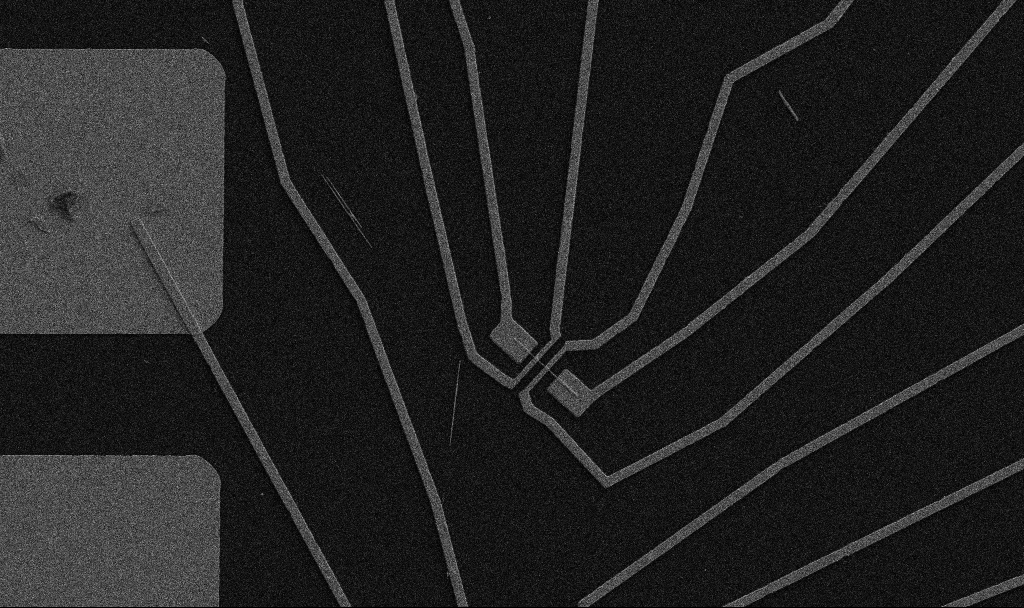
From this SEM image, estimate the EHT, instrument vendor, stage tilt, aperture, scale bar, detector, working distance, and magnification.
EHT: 5 kV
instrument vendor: Zeiss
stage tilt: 0°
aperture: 30 µm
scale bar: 10000 nm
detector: SE2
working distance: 10.7 mm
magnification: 5 K X